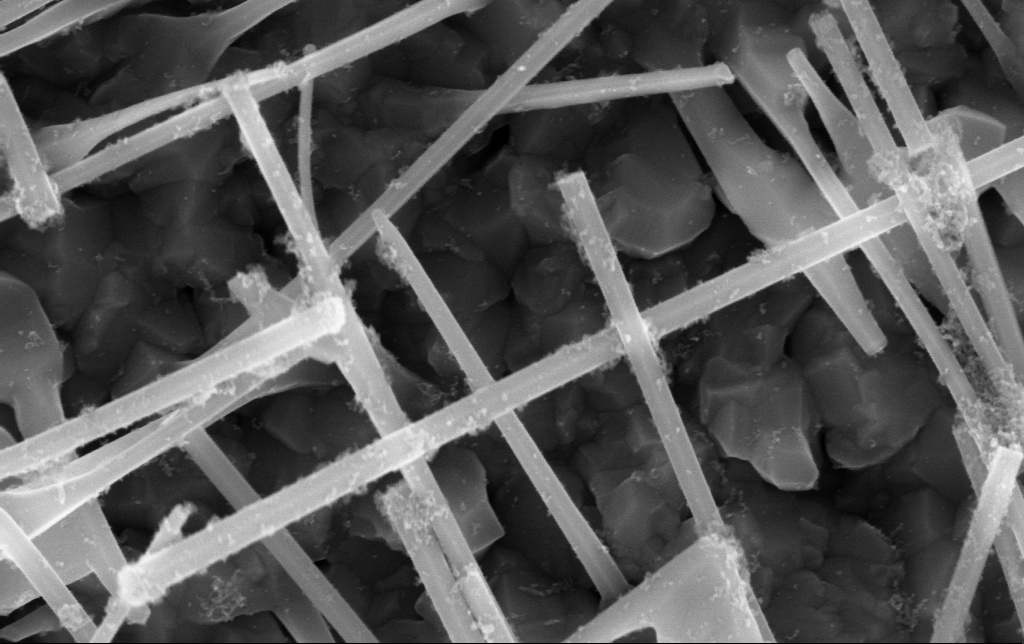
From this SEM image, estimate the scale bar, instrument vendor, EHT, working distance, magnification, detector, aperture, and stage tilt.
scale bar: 100 nm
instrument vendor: Zeiss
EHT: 10 kV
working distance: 8.1 mm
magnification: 120 K X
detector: InLens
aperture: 30 µm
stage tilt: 0.1°